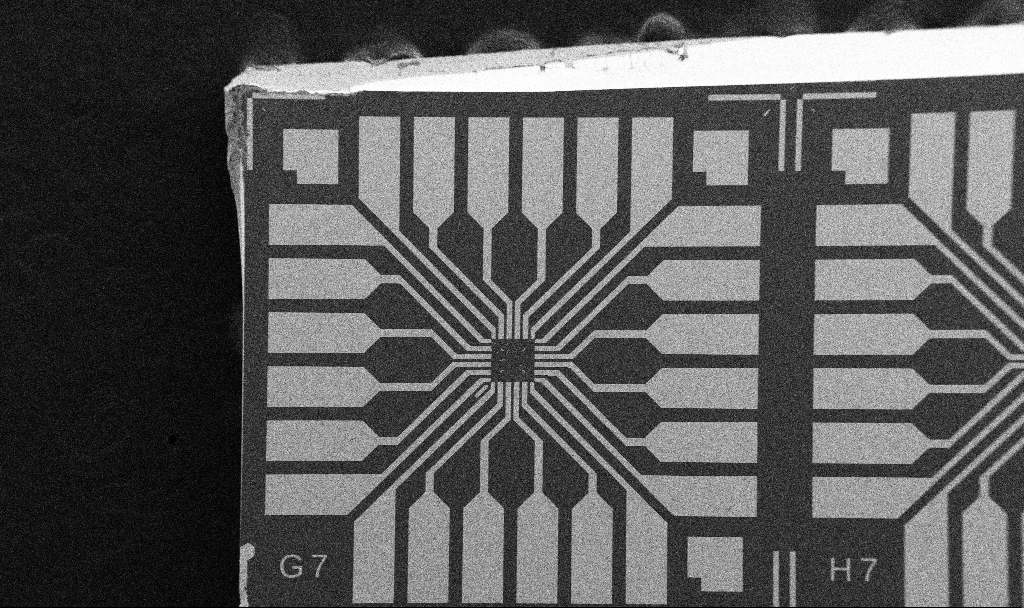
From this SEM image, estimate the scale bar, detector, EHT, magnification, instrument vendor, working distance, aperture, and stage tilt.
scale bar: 200000 nm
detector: SE2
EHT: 5 kV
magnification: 0.1 K X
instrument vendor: Zeiss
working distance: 10.7 mm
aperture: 30 µm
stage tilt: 0°